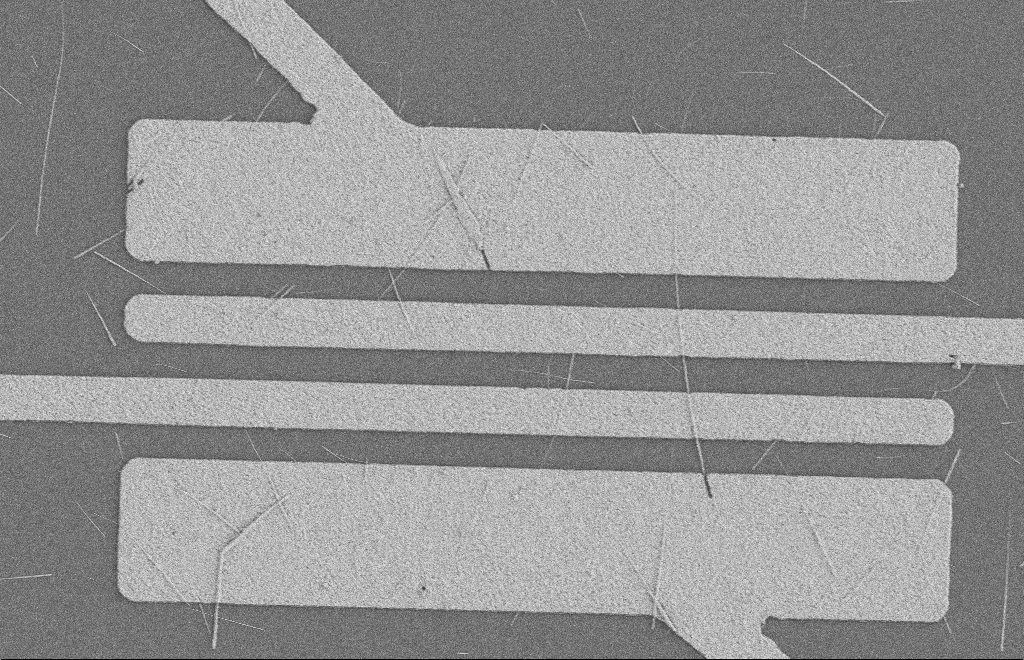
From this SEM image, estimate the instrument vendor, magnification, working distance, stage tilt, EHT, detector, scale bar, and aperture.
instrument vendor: Zeiss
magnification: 5.01 K X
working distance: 8 mm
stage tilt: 0°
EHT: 2 kV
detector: SE2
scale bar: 2000 nm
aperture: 20 µm